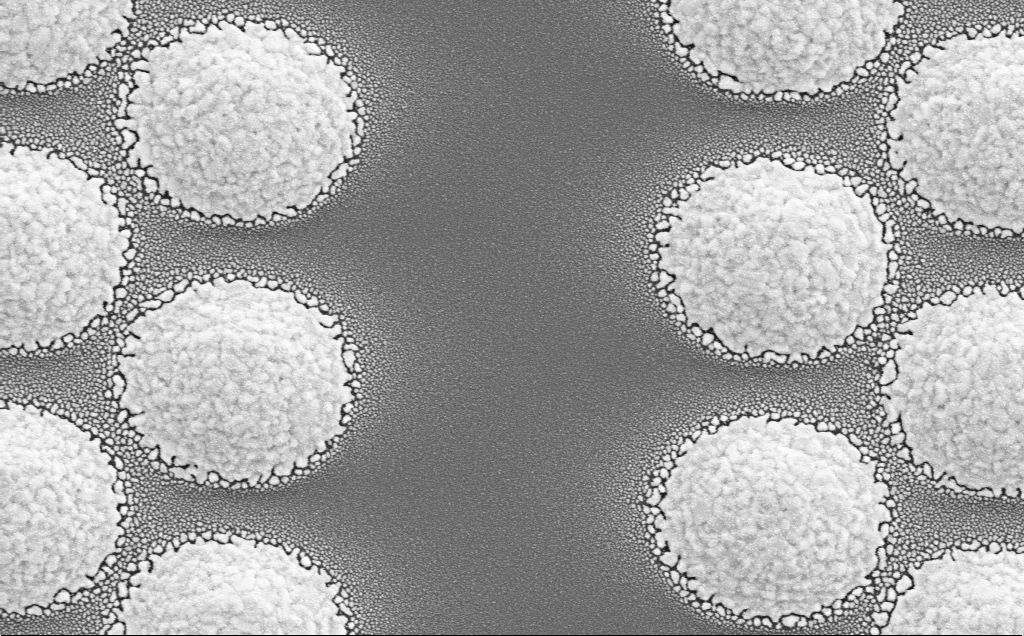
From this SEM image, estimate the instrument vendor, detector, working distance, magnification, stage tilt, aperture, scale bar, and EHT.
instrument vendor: Zeiss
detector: SE2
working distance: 20 mm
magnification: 31.54 K X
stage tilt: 0°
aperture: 30 µm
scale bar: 2000 nm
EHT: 5 kV